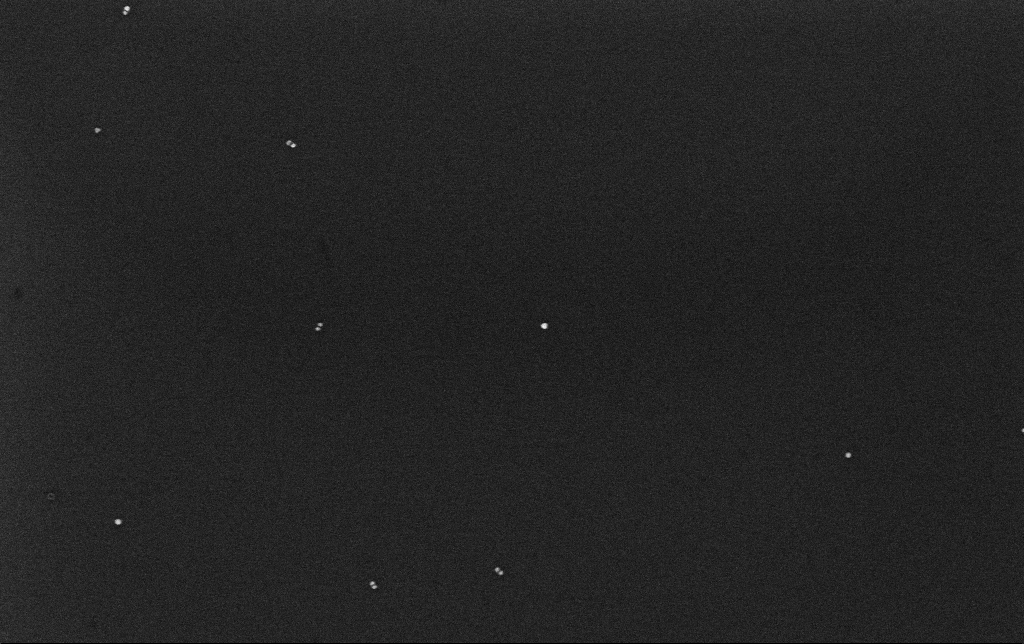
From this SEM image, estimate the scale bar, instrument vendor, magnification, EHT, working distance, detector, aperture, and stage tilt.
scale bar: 200 nm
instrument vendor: Zeiss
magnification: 100 K X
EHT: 10 kV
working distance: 3.2 mm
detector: InLens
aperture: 30 µm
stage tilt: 0°